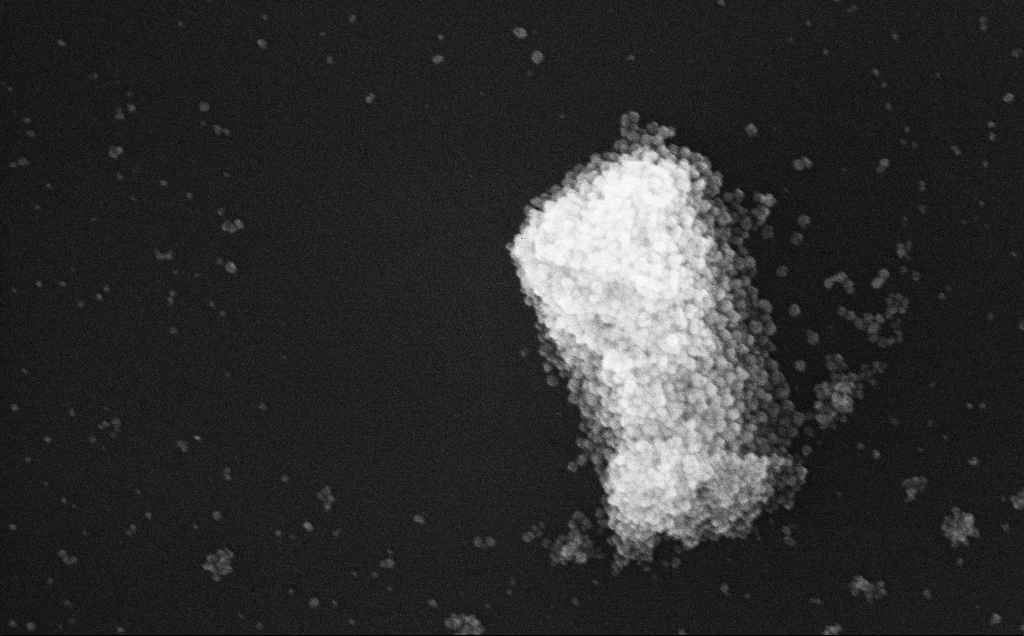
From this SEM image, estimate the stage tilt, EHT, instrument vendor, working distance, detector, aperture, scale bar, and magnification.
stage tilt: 0°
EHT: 10 kV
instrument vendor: Zeiss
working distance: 3.7 mm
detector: InLens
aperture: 30 µm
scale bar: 200 nm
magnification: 176.54 K X